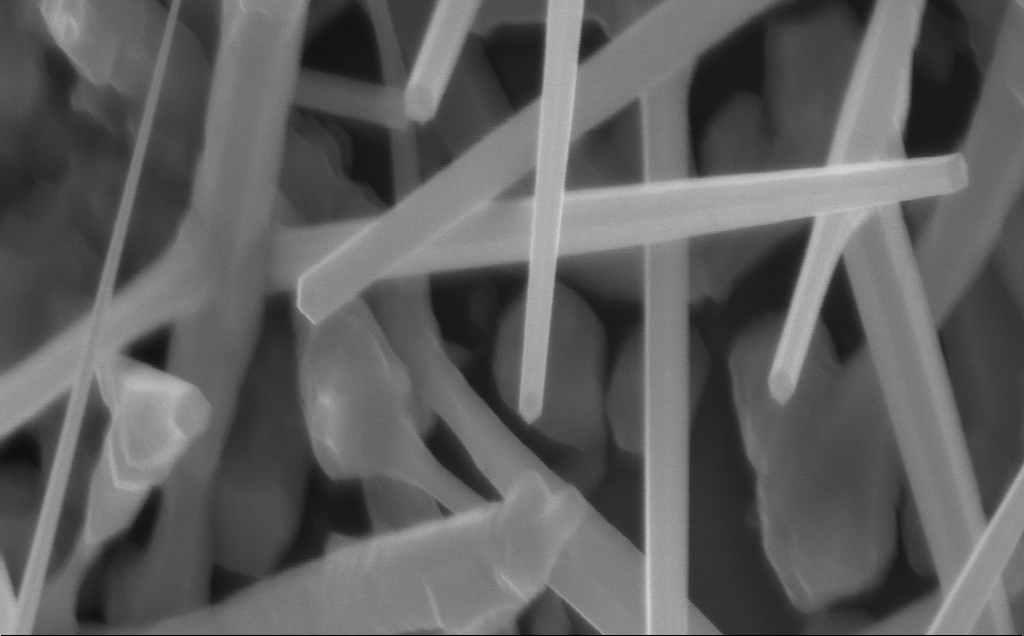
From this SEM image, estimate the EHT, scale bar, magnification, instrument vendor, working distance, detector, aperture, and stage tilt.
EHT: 10 kV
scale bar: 200 nm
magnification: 80 K X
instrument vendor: Zeiss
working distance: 4 mm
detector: InLens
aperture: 30 µm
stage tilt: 0°